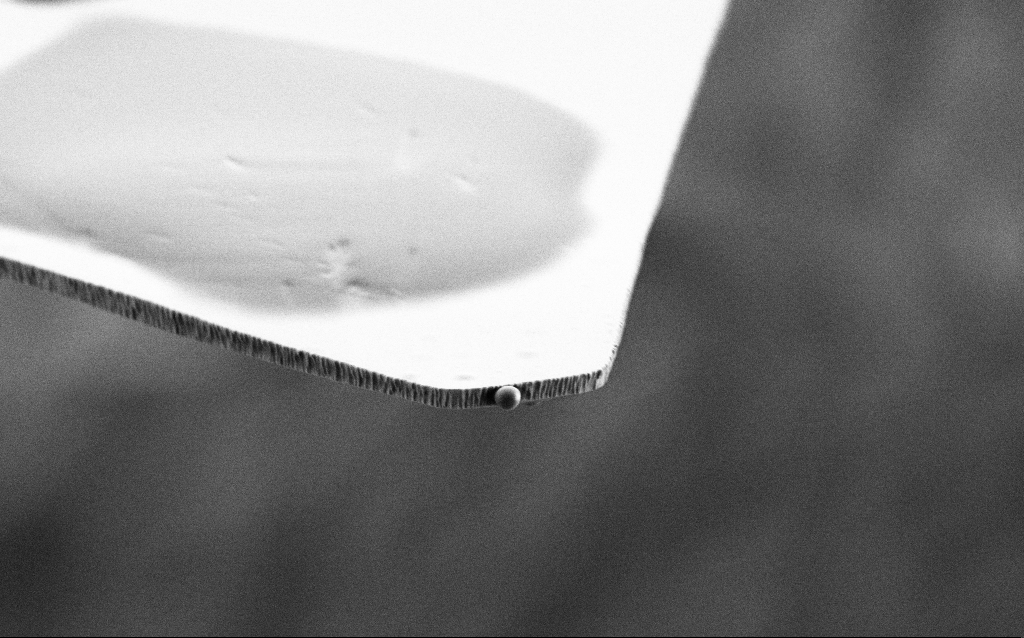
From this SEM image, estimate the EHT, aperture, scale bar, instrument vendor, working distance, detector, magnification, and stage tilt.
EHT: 3 kV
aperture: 30 µm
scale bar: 2000 nm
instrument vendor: Zeiss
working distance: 6 mm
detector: SE2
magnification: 10.13 K X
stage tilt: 70°